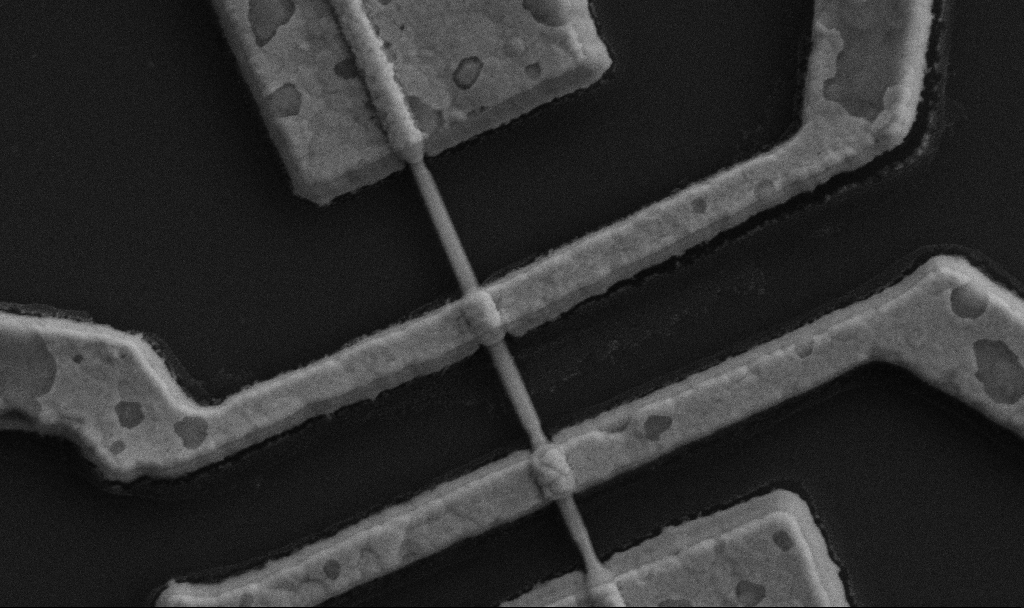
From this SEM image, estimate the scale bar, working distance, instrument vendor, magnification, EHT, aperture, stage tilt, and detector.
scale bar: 1000 nm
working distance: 9.7 mm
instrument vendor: Zeiss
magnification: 60 K X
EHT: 5 kV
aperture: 30 µm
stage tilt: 0°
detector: SE2